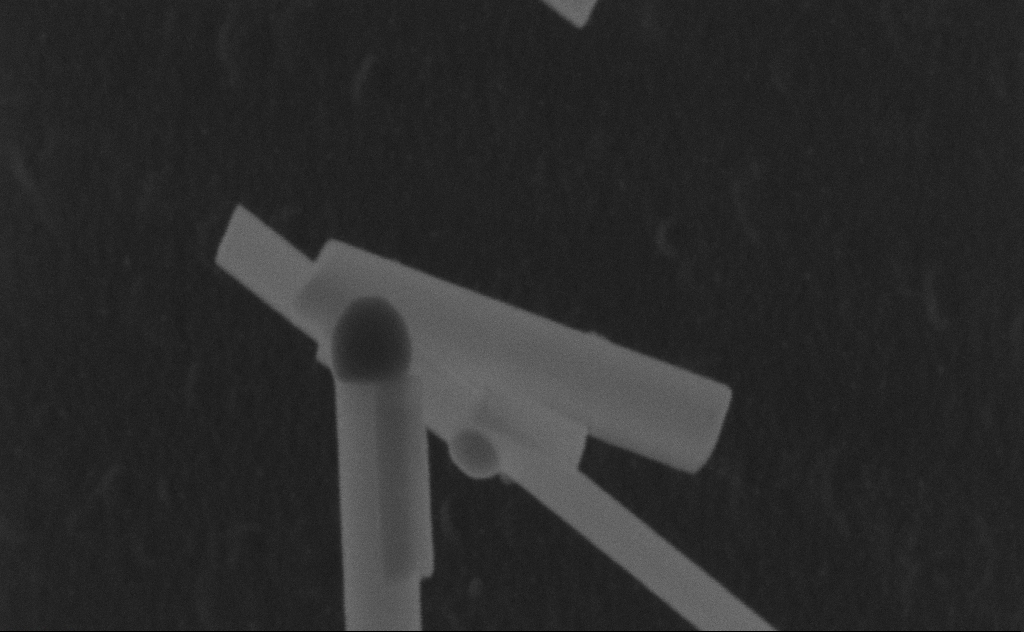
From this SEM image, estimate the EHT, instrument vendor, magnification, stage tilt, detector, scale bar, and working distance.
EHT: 20 kV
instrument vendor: Zeiss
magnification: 301.72 K X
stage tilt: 0°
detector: SE2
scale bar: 200 nm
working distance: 8 mm